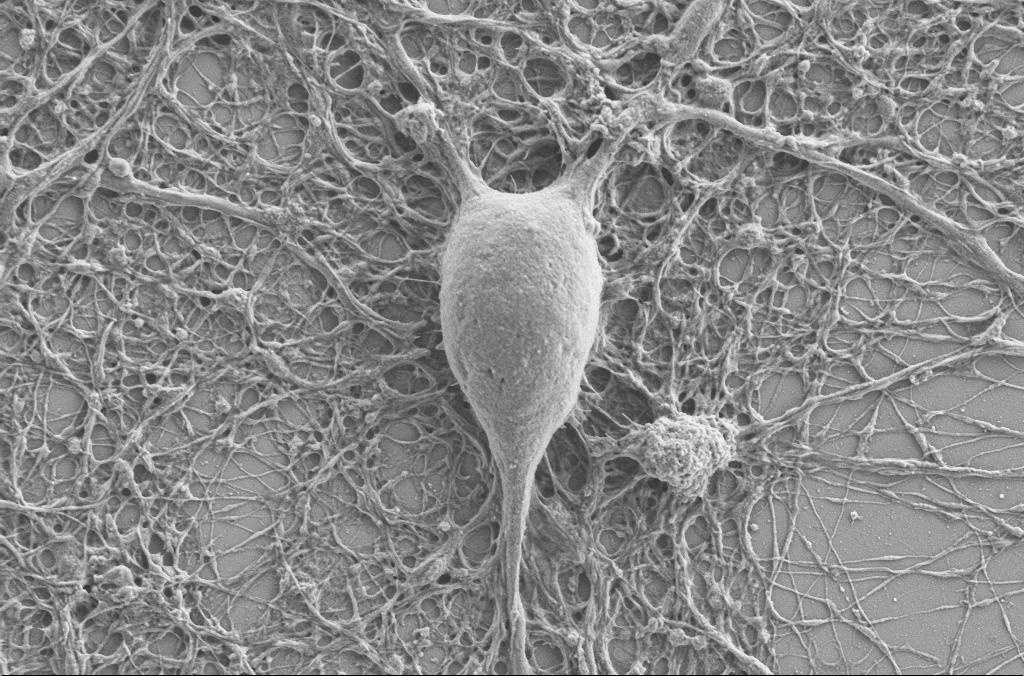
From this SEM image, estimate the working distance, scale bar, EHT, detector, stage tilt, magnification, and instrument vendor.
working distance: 4 mm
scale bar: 2000 nm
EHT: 2 kV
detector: SE2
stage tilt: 0°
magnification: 10 K X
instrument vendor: Zeiss